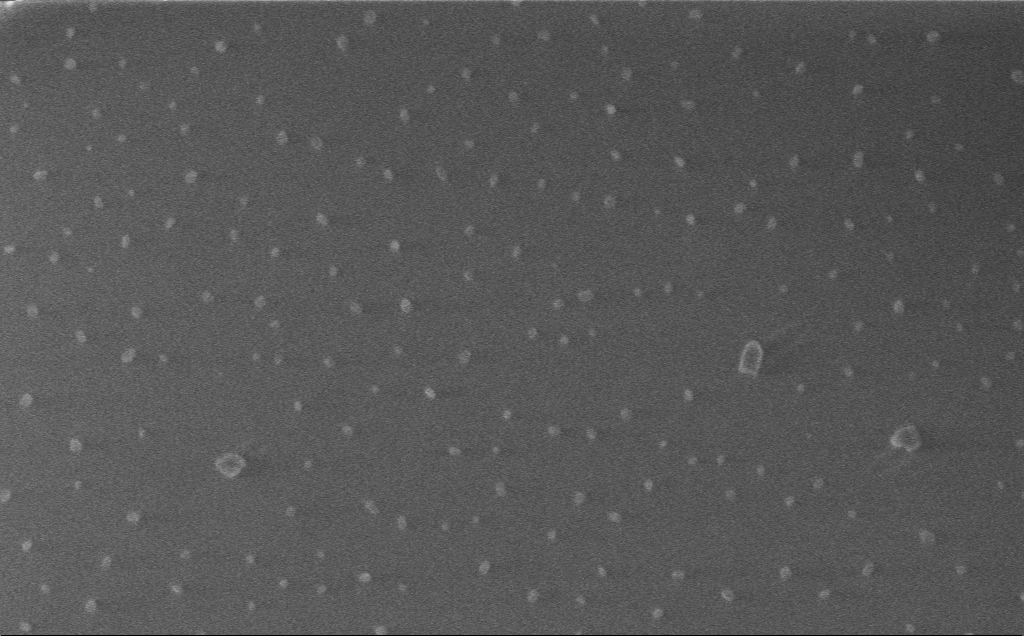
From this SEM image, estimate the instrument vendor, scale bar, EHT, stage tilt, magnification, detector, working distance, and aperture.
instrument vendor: Zeiss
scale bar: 1000 nm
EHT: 1 kV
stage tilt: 0°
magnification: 55.76 K X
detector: InLens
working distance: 3 mm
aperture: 30 µm